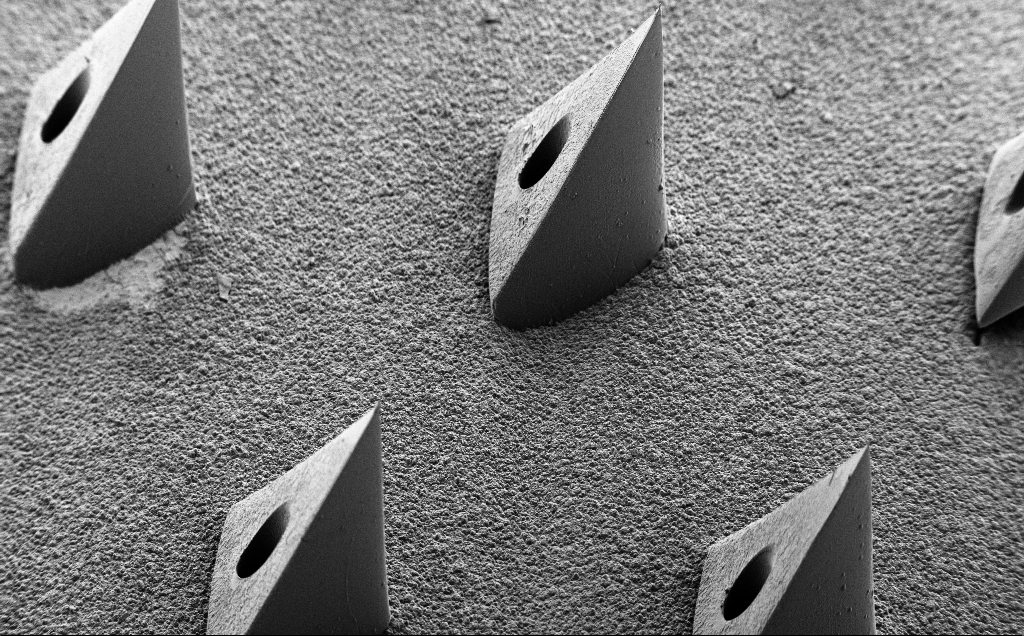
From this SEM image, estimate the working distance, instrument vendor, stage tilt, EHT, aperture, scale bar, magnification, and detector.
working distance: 9 mm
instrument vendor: Zeiss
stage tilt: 40°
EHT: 5 kV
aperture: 30 µm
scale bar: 200000 nm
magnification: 0.118 K X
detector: SE2